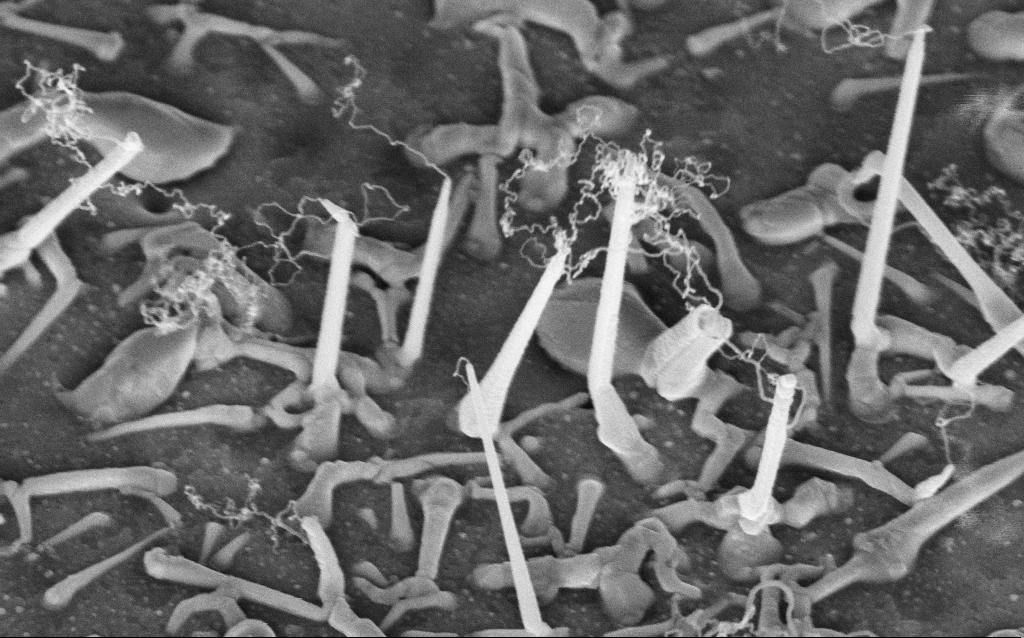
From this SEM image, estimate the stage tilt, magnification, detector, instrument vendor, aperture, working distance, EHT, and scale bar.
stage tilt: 42°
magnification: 67.74 K X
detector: InLens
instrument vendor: Zeiss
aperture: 30 µm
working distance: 8 mm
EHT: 5 kV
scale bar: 1000 nm